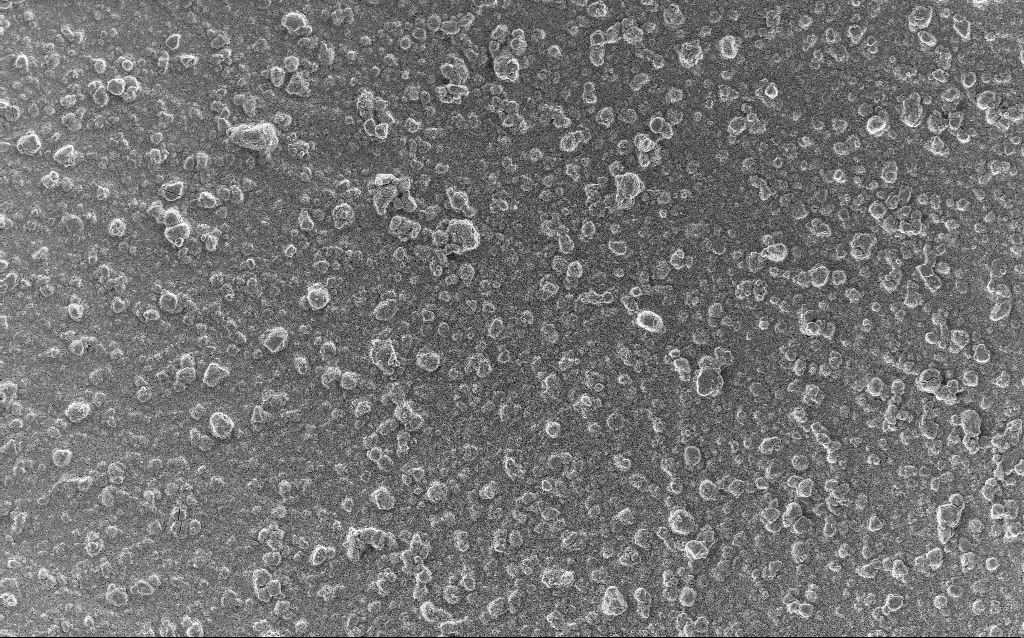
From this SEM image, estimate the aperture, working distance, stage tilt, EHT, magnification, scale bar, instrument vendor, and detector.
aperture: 30 µm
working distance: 2.9 mm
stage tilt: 0°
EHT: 5 kV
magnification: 0.77 K X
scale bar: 20000 nm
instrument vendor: Zeiss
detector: InLens